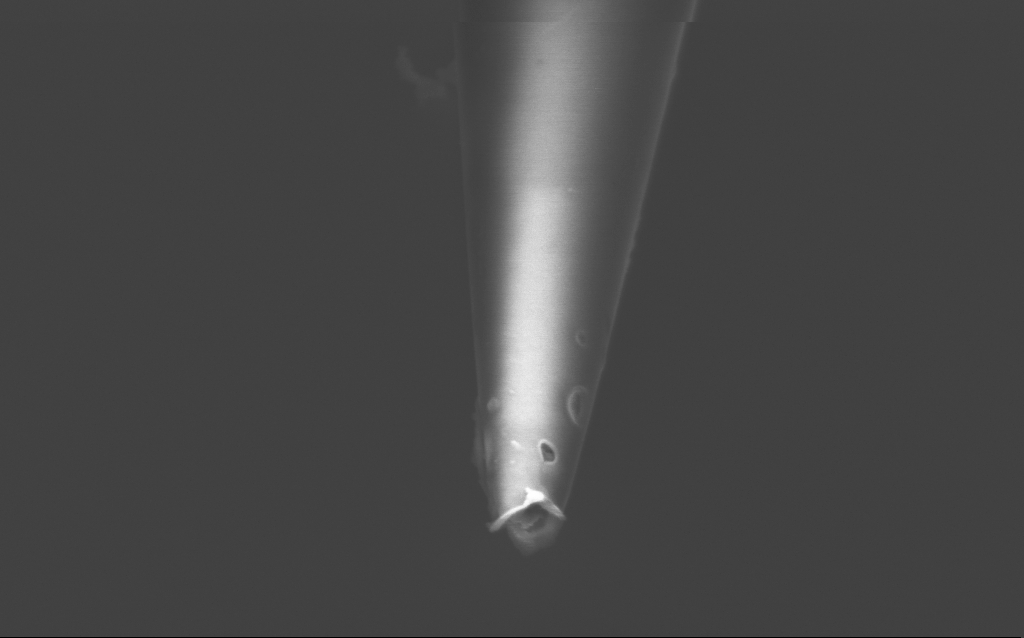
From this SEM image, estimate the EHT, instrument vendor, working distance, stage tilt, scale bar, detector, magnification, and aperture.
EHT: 2 kV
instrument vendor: Zeiss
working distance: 6 mm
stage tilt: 45°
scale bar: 200 nm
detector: InLens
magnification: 100 K X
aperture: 30 µm